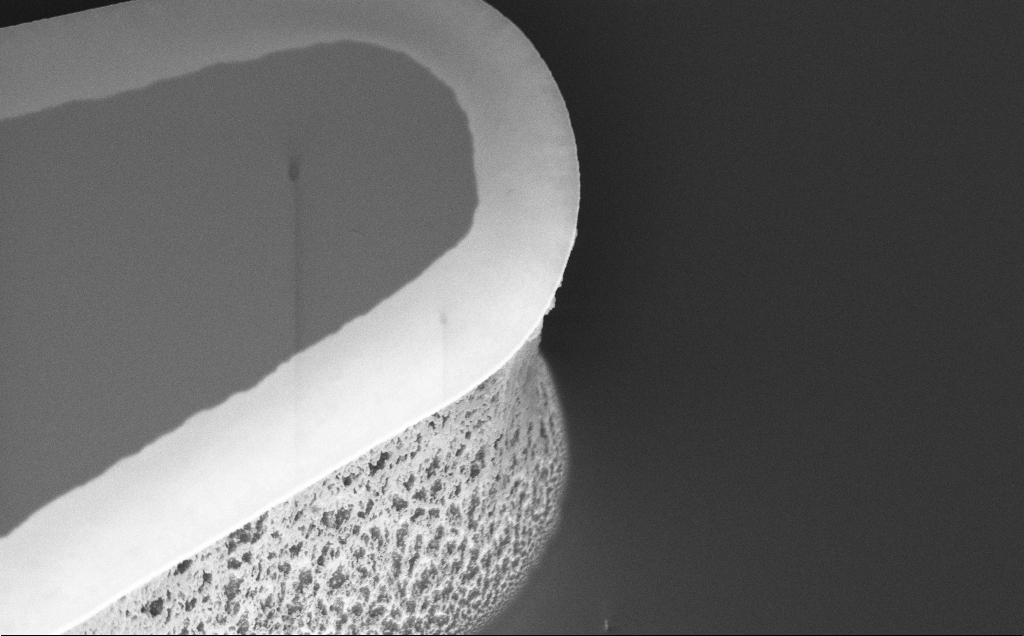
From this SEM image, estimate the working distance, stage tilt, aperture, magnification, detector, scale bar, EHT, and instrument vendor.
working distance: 8 mm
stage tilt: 45°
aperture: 30 µm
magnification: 7.94 K X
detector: InLens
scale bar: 2000 nm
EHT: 5 kV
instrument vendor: Zeiss